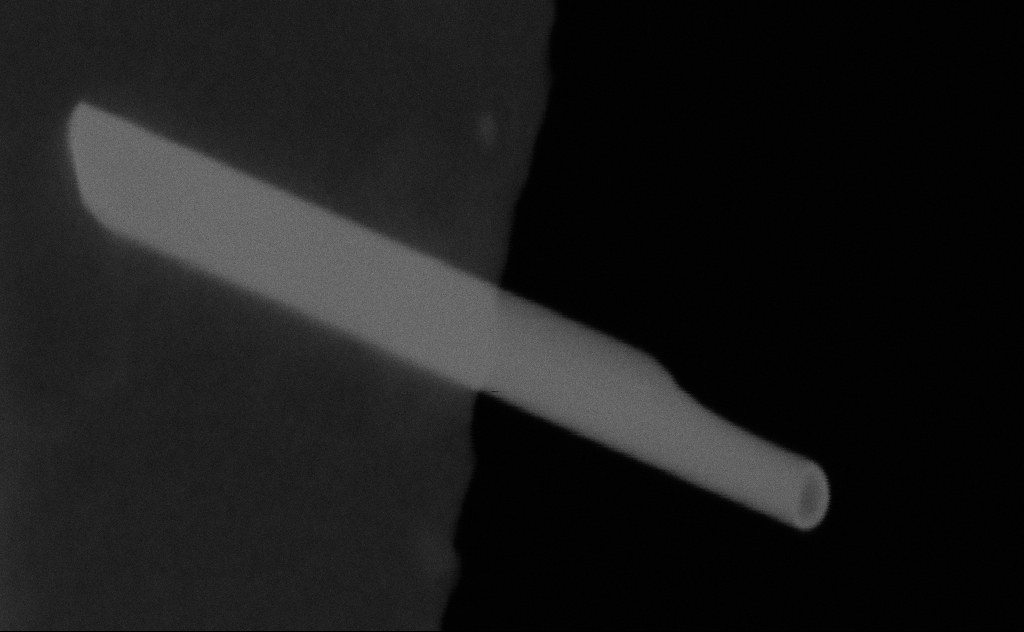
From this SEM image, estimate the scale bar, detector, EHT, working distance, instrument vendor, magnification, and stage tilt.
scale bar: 100 nm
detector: SE2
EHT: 20 kV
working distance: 9 mm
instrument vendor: Zeiss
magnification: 493.08 K X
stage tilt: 0°